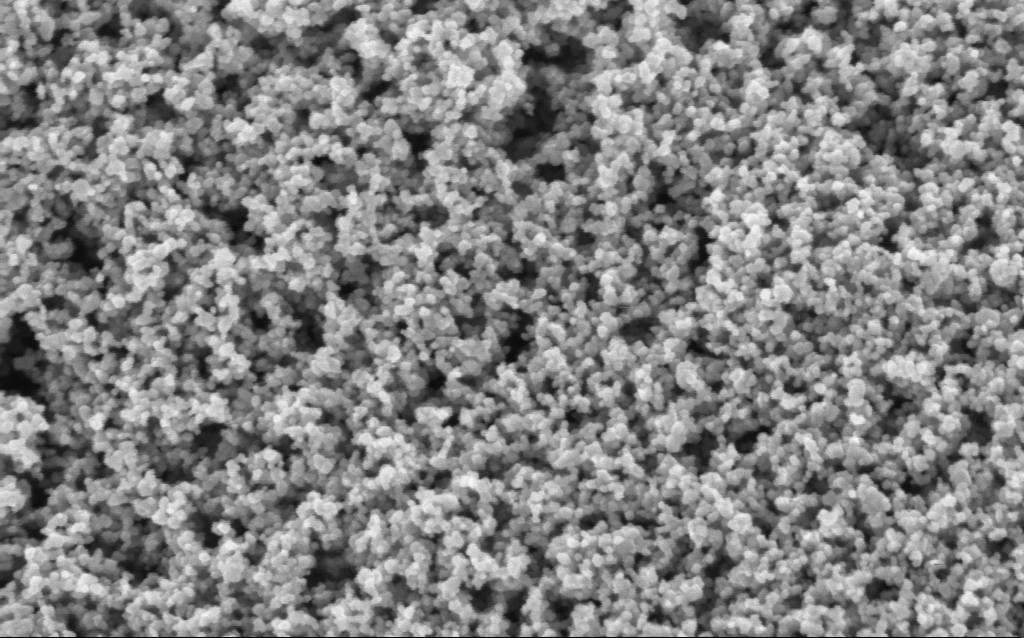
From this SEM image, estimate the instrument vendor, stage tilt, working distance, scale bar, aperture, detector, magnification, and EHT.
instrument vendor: Zeiss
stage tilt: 0°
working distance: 7.6 mm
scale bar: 100 nm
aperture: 30 µm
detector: InLens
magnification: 130 K X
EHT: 3 kV